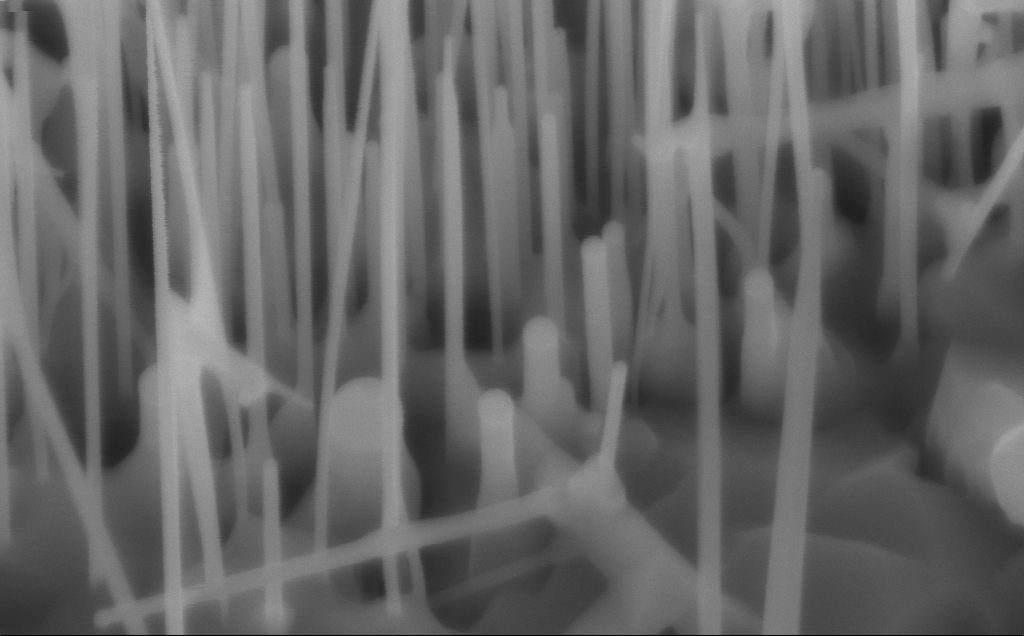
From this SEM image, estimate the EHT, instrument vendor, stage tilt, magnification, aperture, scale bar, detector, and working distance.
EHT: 10 kV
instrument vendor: Zeiss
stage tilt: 30°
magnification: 150 K X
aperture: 30 µm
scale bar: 100 nm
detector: InLens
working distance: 5 mm